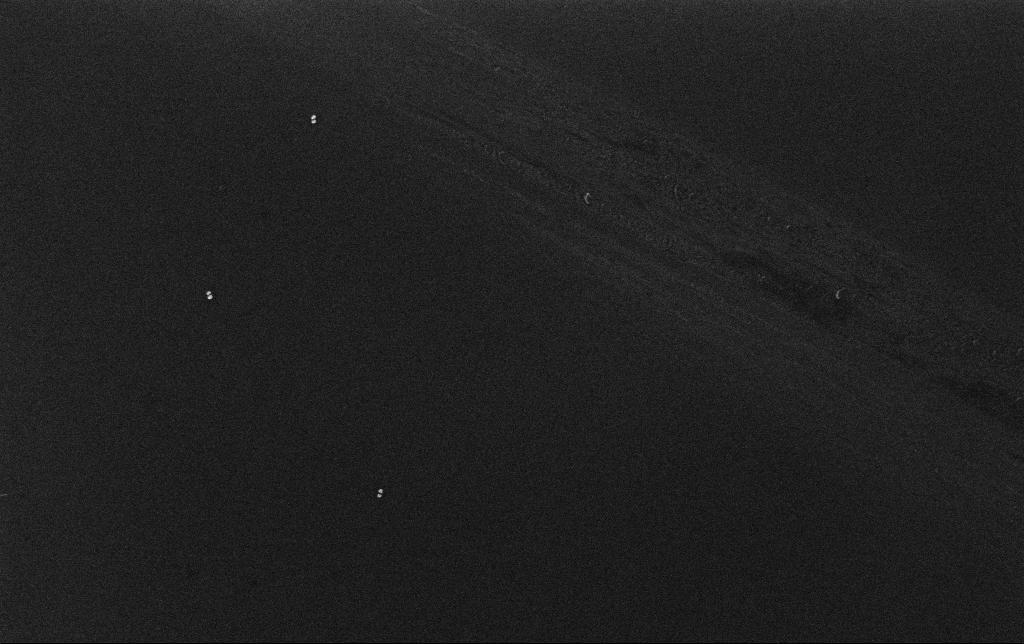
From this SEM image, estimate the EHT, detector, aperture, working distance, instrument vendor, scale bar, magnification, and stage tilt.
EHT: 10 kV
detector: InLens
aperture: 30 µm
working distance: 3.2 mm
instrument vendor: Zeiss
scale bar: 200 nm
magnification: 100 K X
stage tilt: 0°